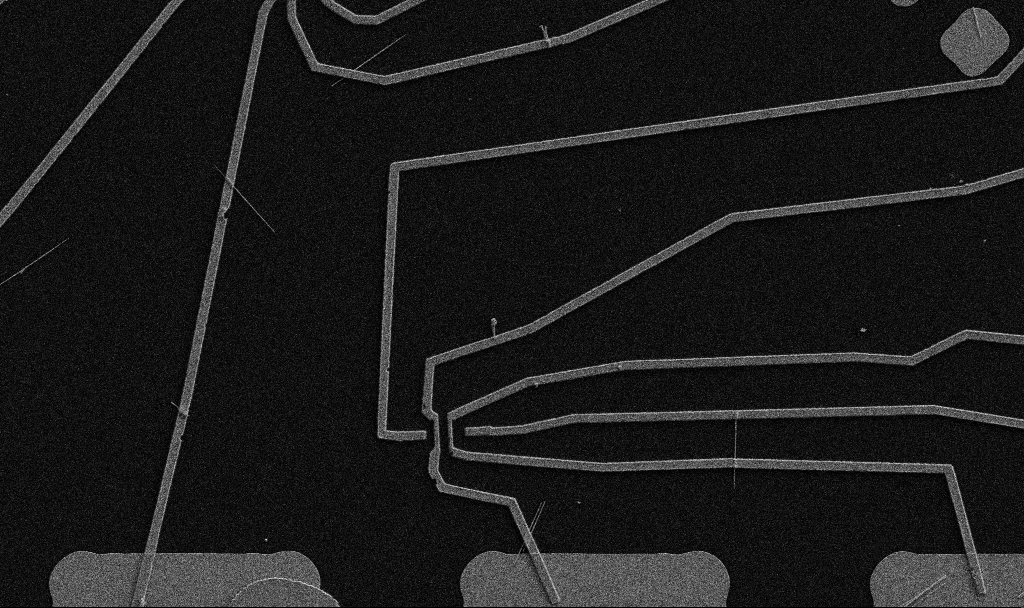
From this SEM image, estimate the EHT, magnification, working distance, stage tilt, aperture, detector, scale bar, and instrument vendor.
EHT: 5 kV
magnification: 5 K X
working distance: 10.7 mm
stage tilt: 0°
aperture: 30 µm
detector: SE2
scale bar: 10000 nm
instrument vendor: Zeiss